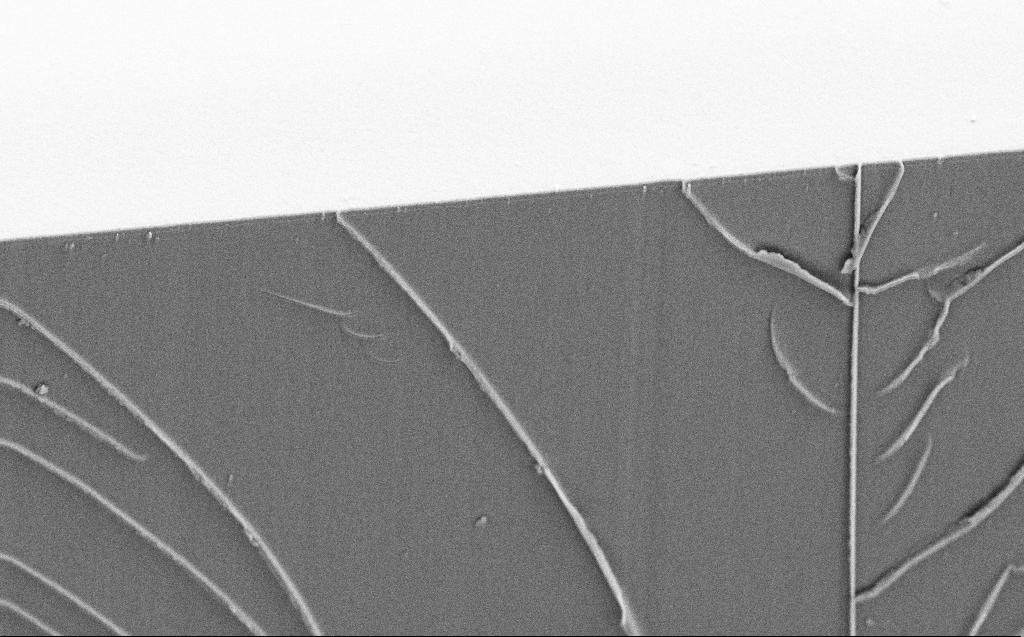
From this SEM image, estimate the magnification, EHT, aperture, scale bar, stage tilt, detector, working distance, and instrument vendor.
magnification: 3.89 K X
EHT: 10 kV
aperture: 30 µm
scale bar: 10000 nm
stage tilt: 45°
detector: SE2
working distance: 5 mm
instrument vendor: Zeiss